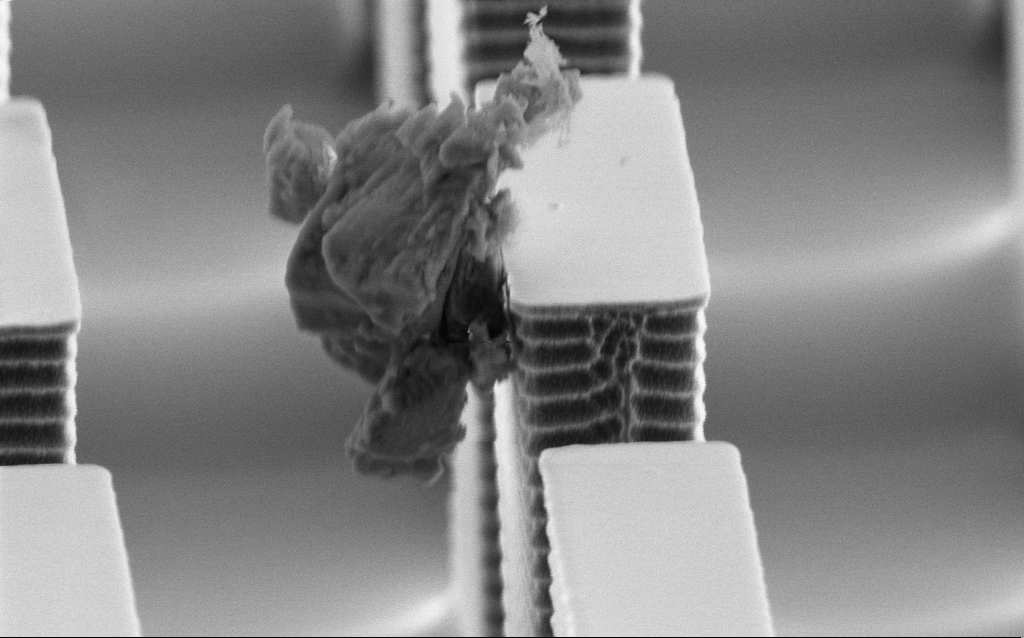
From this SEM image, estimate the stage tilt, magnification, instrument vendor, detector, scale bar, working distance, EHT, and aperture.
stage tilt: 67°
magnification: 40.45 K X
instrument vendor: Zeiss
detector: SE2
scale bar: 1000 nm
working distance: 9.8 mm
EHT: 3 kV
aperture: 30 µm